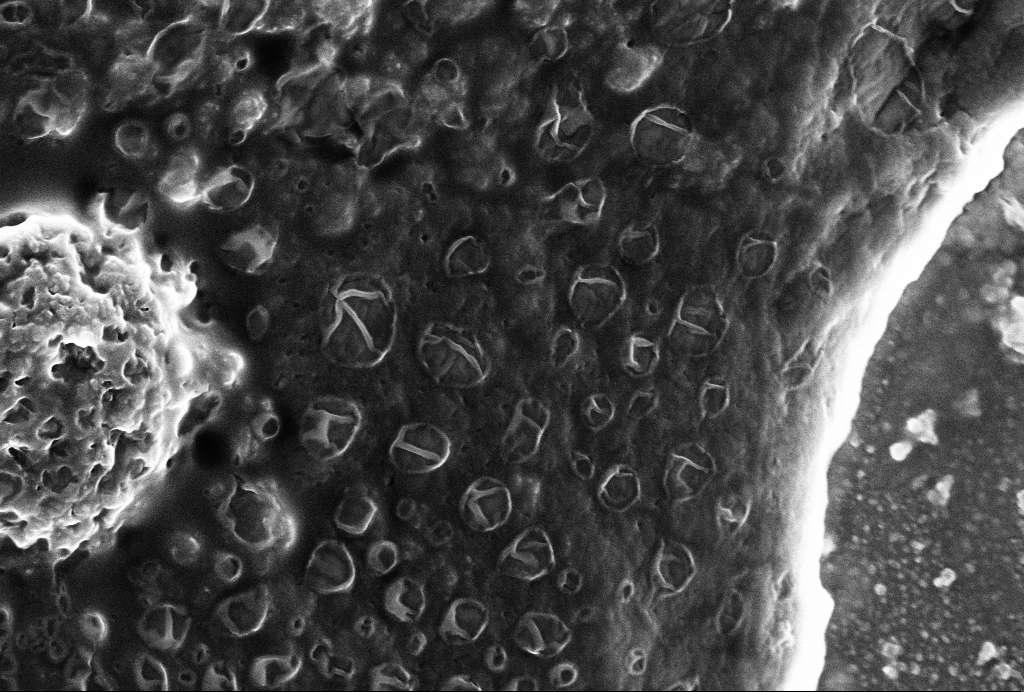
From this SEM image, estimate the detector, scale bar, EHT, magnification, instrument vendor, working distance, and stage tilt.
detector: SE2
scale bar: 1000 nm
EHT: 4 kV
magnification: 50 K X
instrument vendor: Zeiss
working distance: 6 mm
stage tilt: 0°